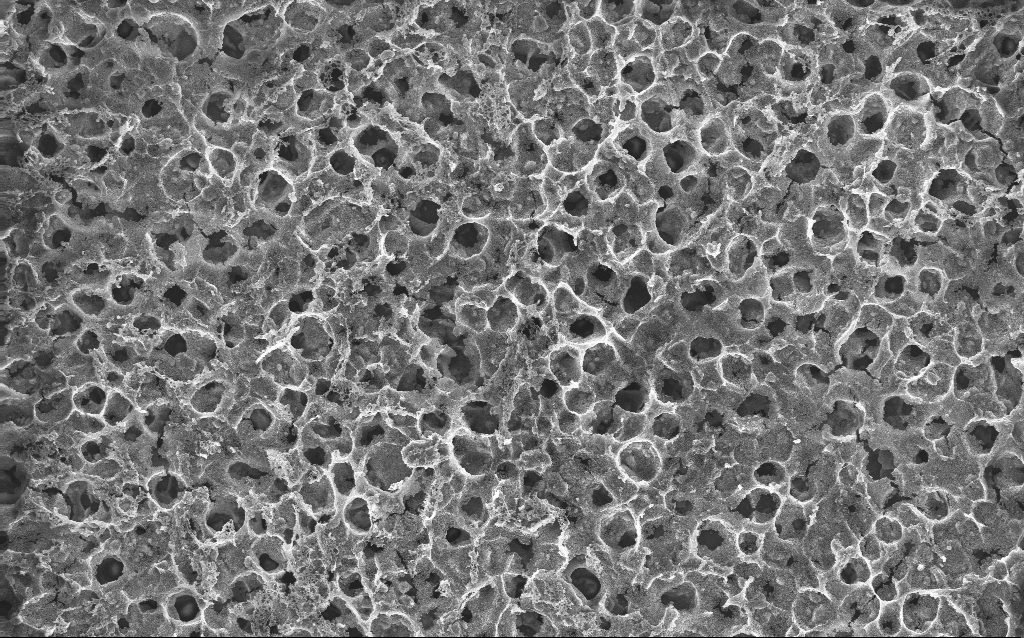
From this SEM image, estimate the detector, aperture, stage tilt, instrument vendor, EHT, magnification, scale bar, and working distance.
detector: InLens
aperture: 30 µm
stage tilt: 0°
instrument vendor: Zeiss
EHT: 10 kV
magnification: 1.69 K X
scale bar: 10000 nm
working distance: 2.4 mm